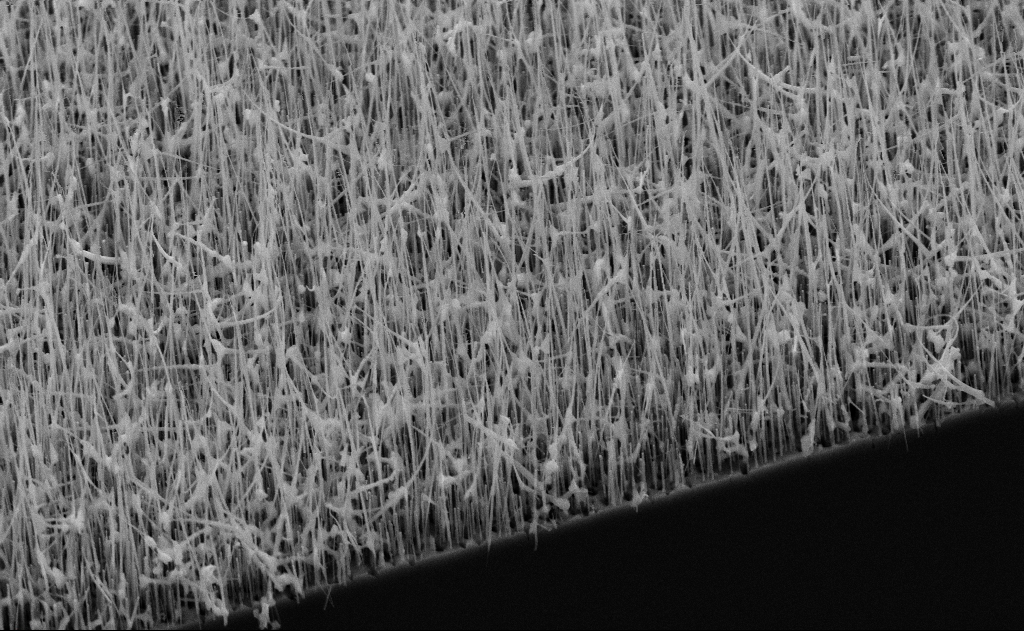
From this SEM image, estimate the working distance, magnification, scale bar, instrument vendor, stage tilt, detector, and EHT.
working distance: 12 mm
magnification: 20 K X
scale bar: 2000 nm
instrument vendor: Zeiss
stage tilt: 45°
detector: SE2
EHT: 10 kV